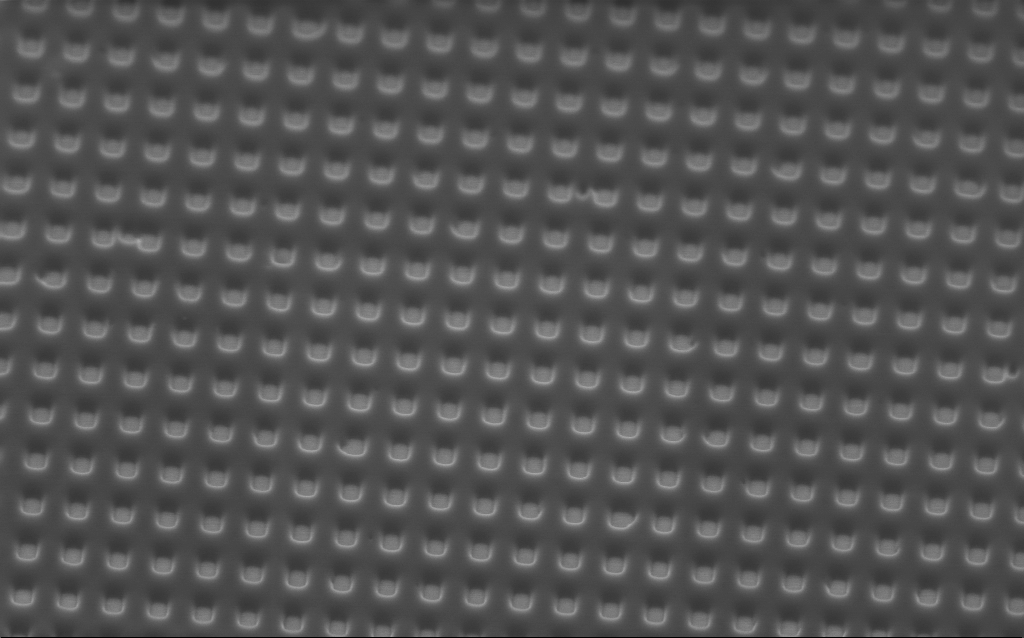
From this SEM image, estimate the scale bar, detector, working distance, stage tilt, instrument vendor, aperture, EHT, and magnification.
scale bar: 1000 nm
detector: InLens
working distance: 2.7 mm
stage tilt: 45°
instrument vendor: Zeiss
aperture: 30 µm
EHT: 2 kV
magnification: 33.34 K X